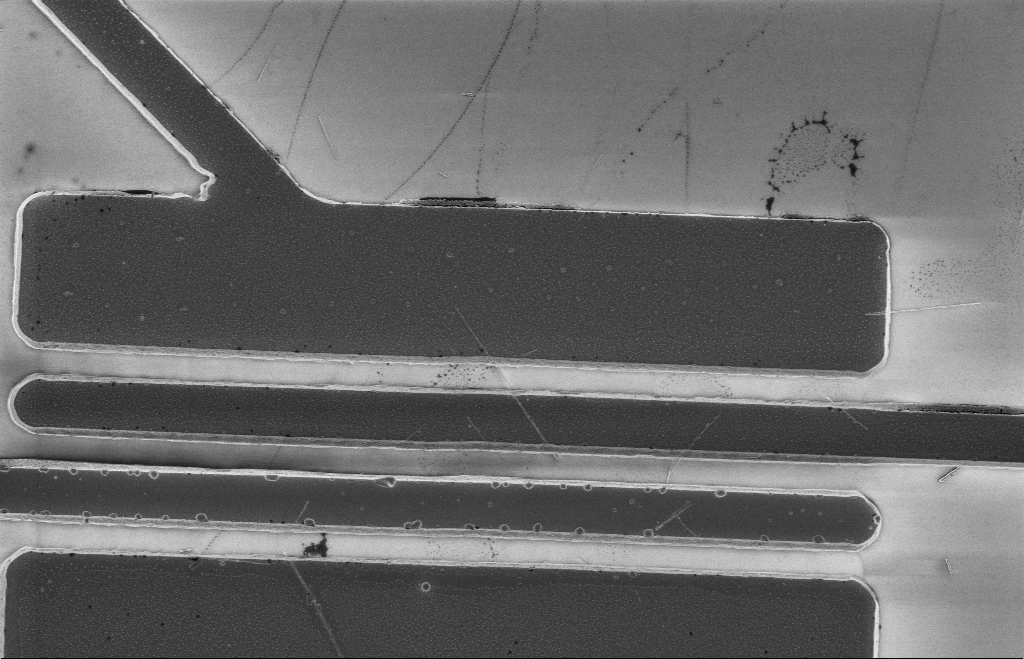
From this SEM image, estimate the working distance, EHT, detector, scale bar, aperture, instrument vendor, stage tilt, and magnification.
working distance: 14 mm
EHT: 5 kV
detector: InLens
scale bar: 2000 nm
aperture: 20 µm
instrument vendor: Zeiss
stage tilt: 0°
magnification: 5.28 K X